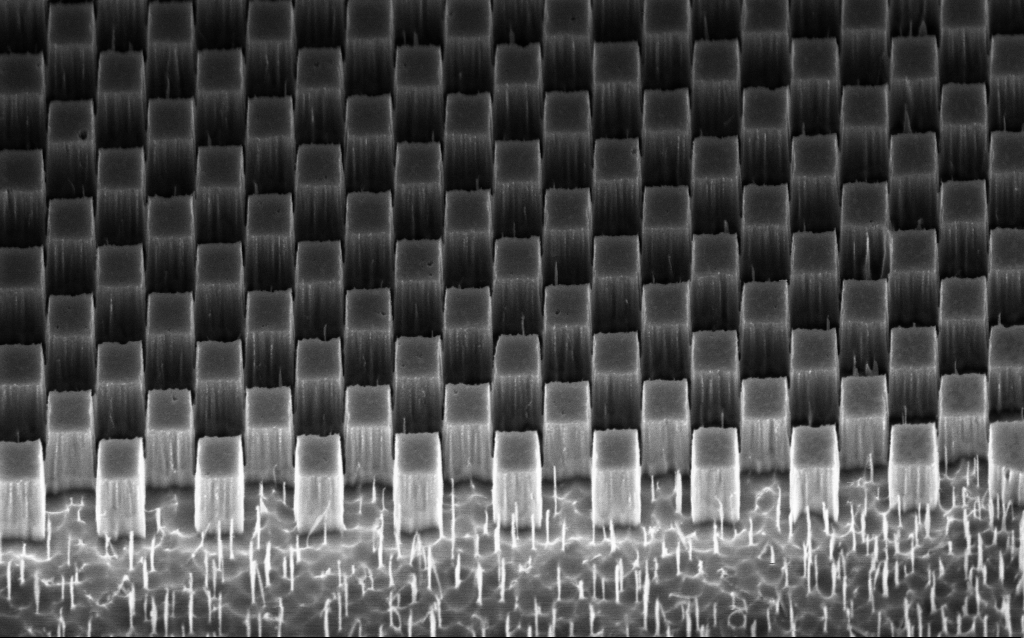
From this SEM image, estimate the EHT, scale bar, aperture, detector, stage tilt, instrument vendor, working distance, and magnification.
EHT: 3 kV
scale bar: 1000 nm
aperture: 30 µm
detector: InLens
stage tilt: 45°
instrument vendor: Zeiss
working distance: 5 mm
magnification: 36.85 K X